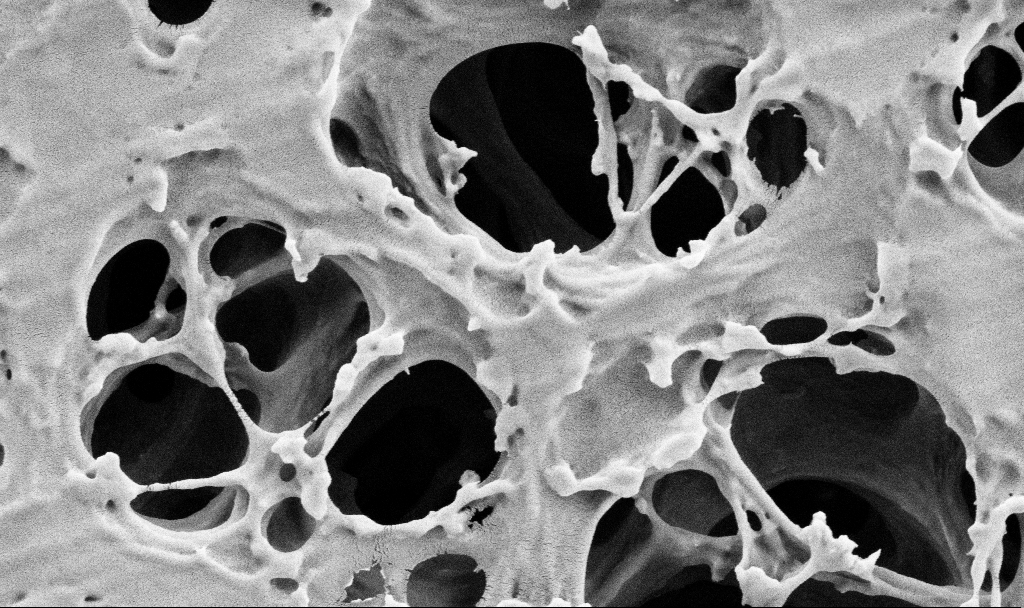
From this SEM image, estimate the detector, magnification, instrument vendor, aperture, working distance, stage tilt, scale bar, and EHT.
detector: SE2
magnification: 50 K X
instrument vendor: Zeiss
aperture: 30 µm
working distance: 3.5 mm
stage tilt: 0°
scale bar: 1000 nm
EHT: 2 kV